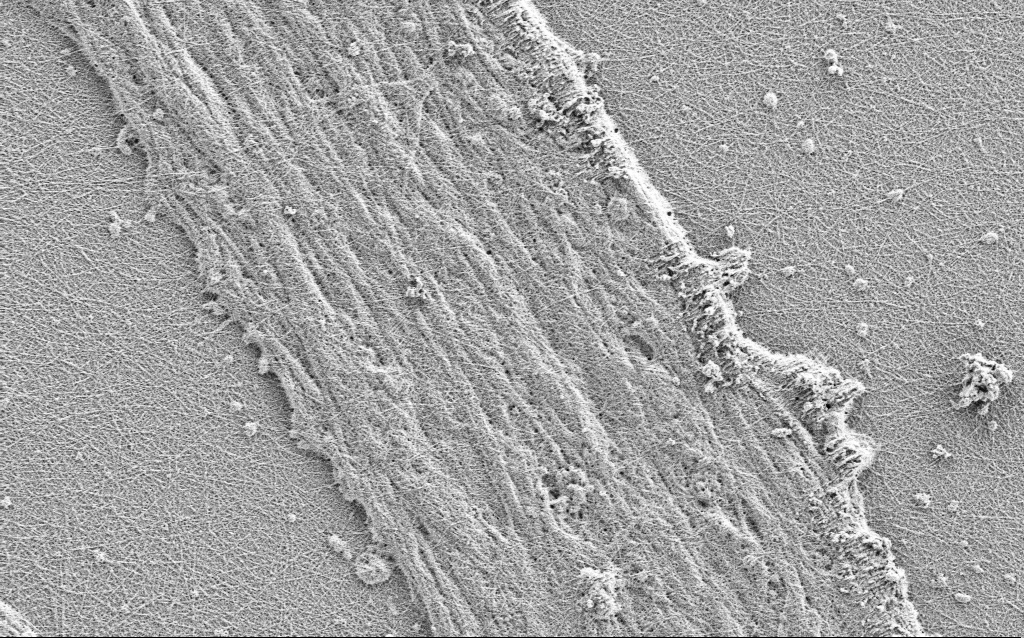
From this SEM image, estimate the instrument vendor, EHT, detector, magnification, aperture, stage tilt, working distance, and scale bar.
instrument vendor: Zeiss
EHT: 0.9 kV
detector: SE2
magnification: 10 K X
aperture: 30 µm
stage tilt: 0°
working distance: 3 mm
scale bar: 2000 nm